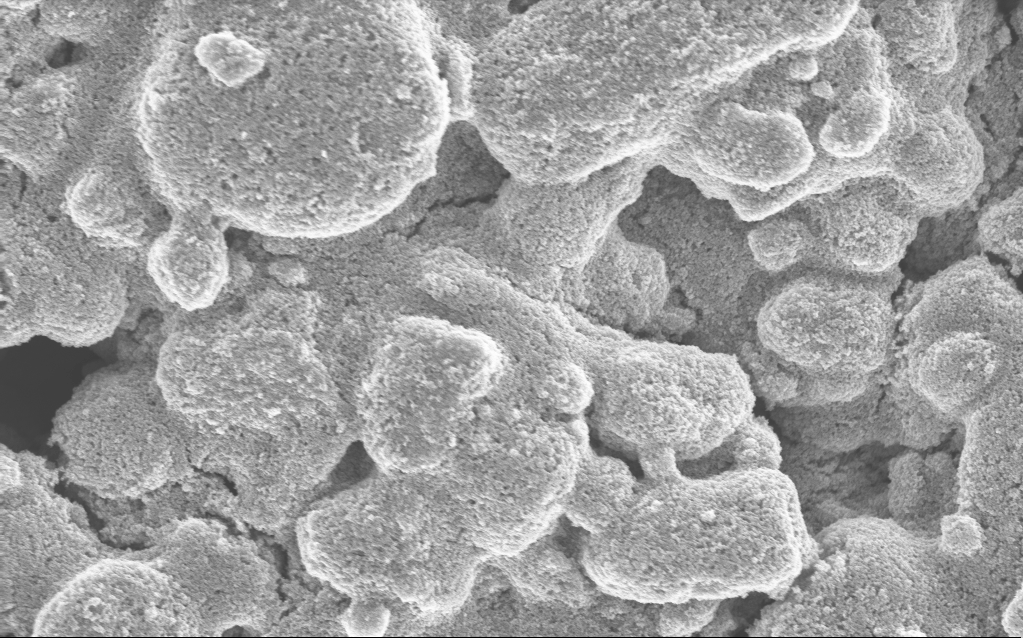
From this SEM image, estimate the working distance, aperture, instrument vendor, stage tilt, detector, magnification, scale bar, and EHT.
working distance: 4.9 mm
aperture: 30 µm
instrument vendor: Zeiss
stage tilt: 0°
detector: InLens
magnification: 21.03 K X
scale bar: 1000 nm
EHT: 5 kV